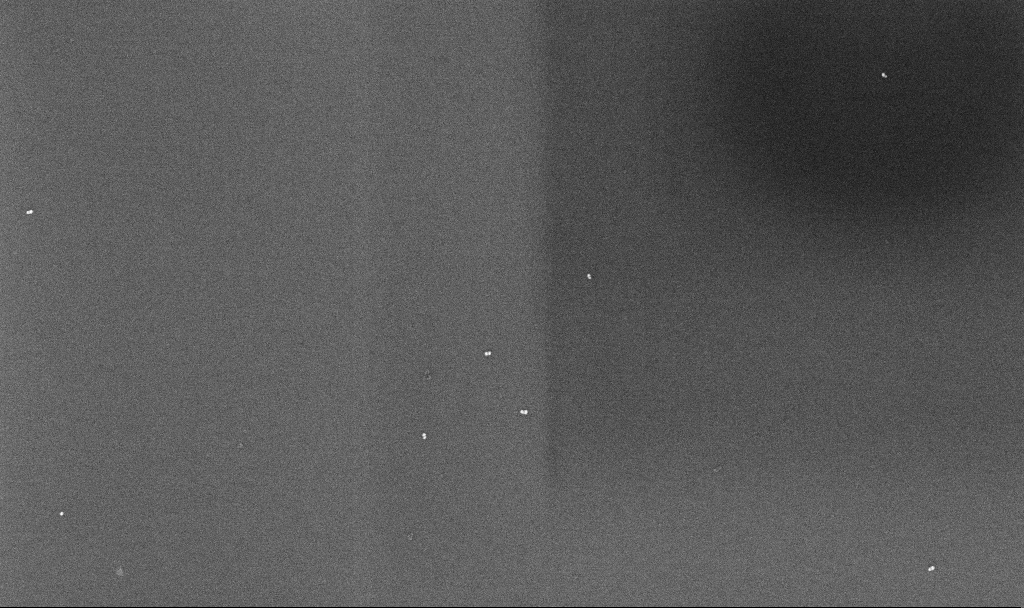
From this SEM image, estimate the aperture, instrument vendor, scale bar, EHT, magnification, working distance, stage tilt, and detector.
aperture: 30 µm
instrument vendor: Zeiss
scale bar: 1000 nm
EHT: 10 kV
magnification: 68.78 K X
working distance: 4.3 mm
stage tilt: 0°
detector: InLens